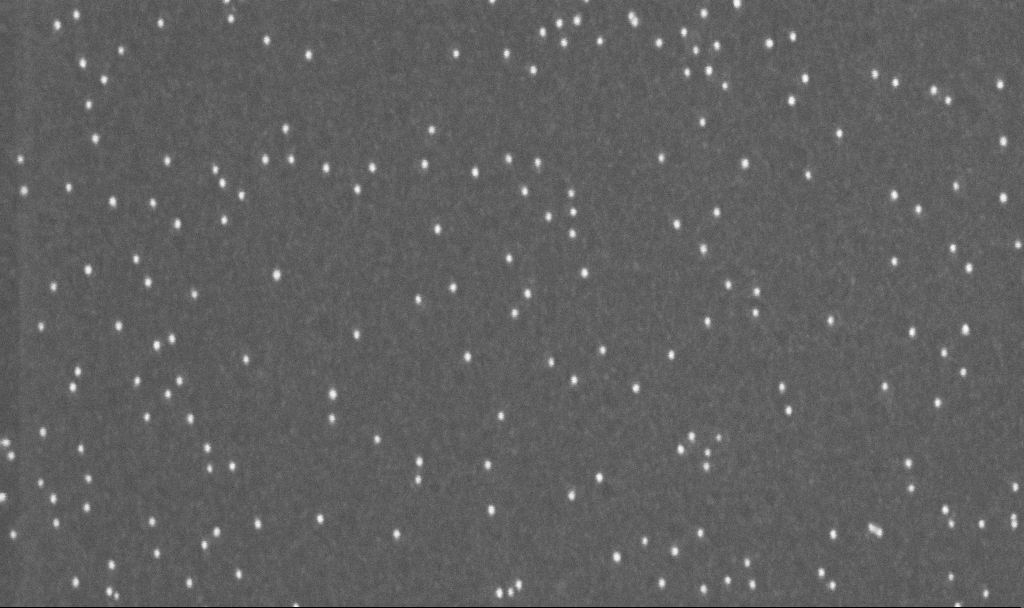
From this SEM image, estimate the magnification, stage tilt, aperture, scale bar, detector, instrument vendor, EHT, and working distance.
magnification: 200 K X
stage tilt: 0°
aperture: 30 µm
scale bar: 100 nm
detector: InLens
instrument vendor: Zeiss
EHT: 10 kV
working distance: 3.2 mm